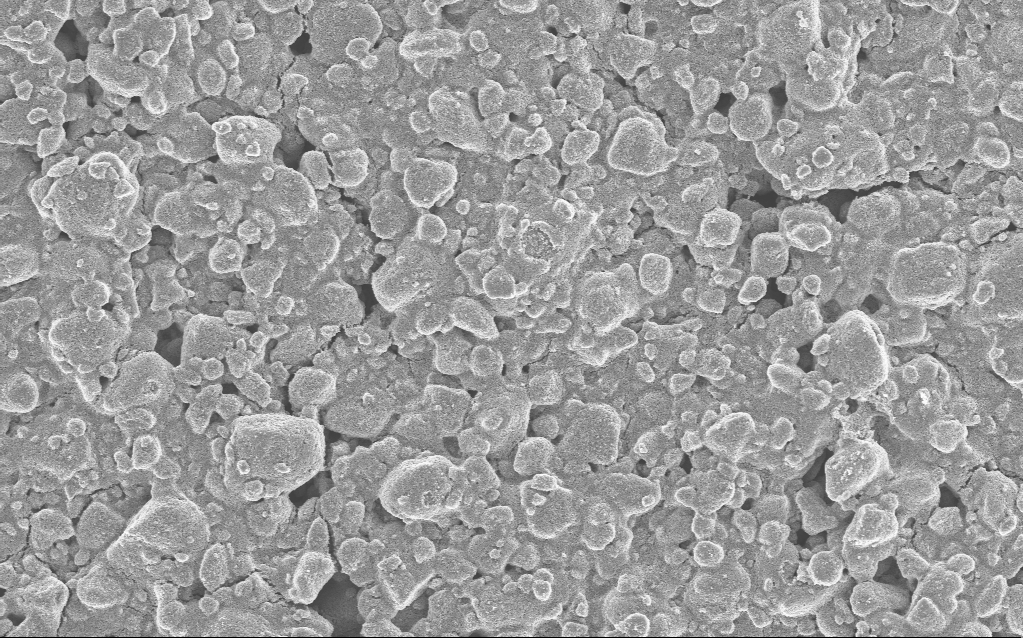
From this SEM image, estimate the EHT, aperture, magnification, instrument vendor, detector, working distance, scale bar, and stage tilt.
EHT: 5 kV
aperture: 30 µm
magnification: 4.39 K X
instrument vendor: Zeiss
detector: InLens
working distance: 4.9 mm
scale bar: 10000 nm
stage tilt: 0°